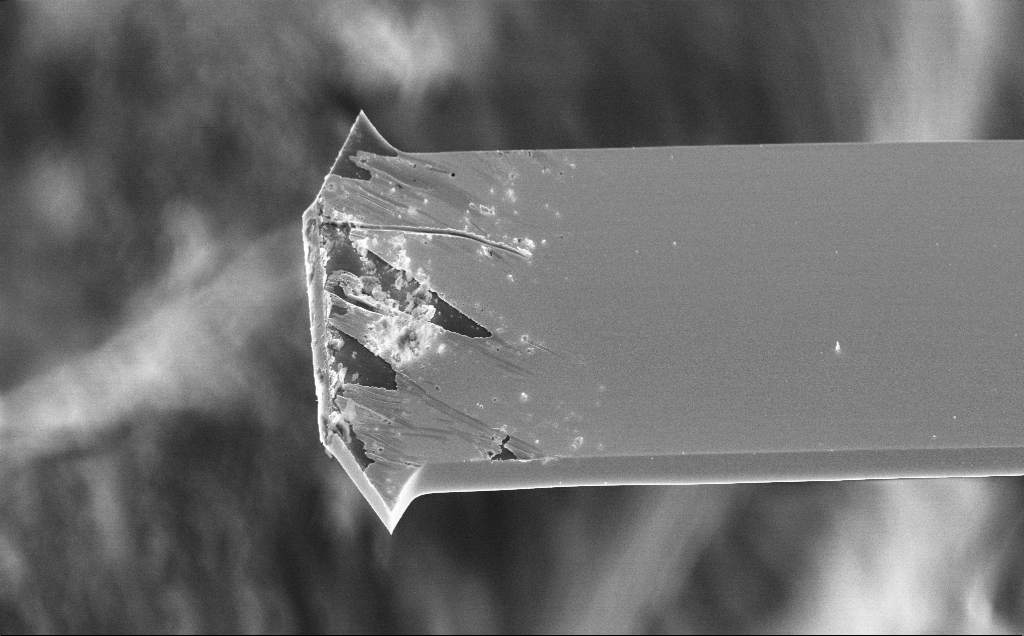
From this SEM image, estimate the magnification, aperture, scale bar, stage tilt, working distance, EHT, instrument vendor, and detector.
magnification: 3.38 K X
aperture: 30 µm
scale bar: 20000 nm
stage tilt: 44.2°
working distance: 3 mm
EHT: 10 kV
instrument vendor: Zeiss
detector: InLens